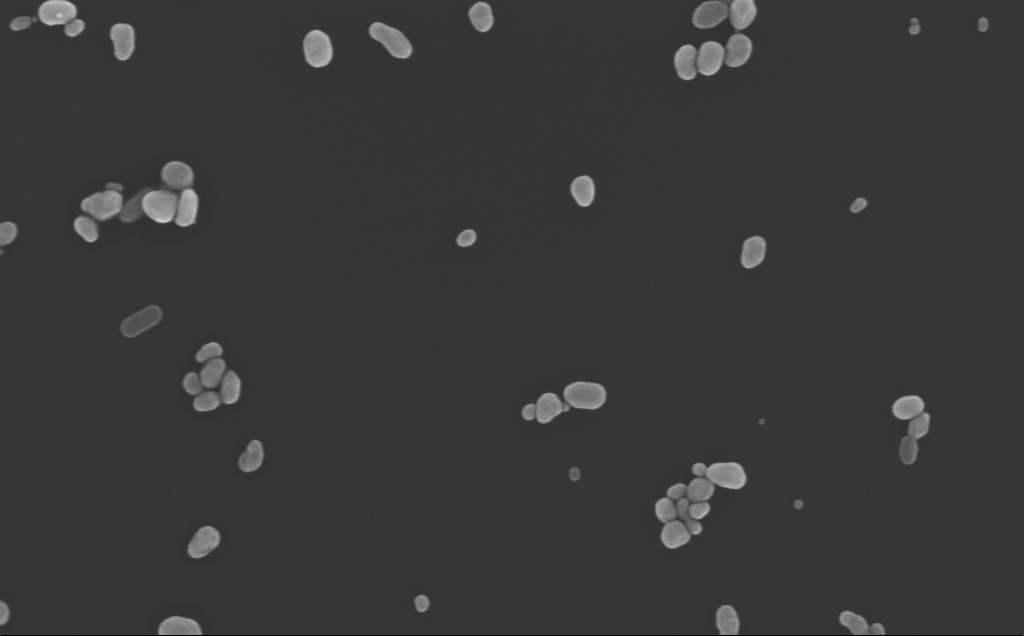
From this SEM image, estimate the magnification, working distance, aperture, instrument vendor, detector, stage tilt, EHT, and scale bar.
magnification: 135.16 K X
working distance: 5 mm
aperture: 30 µm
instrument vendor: Zeiss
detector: InLens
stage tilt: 0°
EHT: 10 kV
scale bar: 200 nm